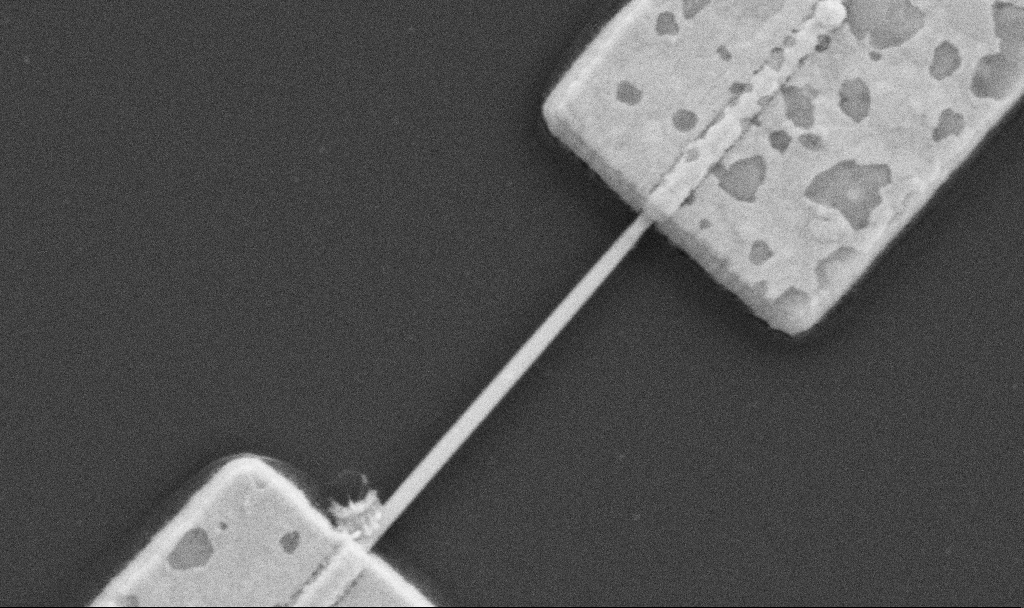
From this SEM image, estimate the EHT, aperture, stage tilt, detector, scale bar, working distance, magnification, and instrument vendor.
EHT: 5 kV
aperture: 30 µm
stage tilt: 0°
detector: SE2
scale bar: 1000 nm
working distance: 10.7 mm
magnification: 60 K X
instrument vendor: Zeiss